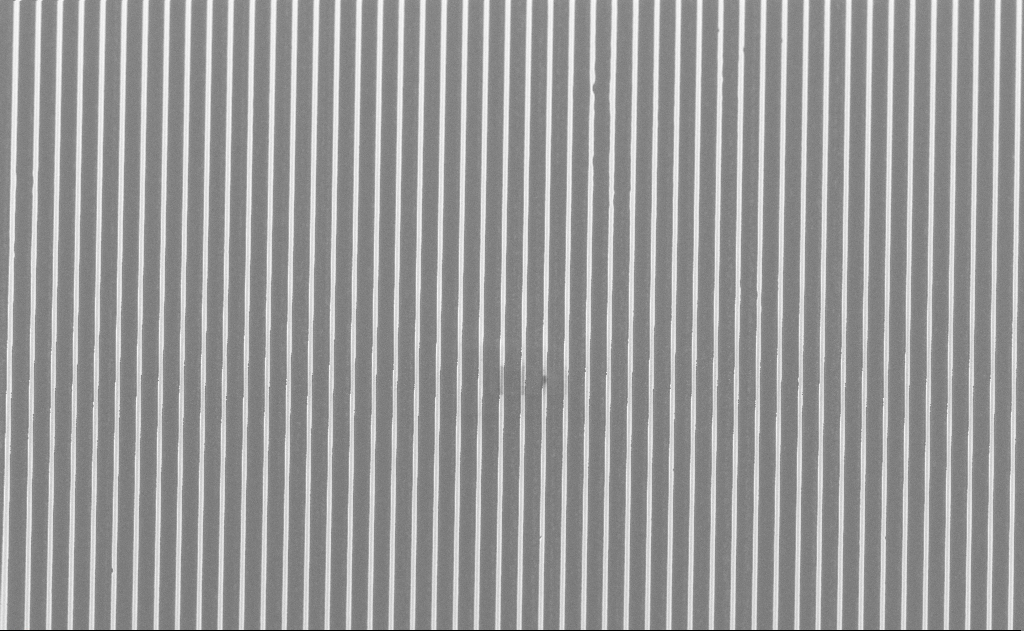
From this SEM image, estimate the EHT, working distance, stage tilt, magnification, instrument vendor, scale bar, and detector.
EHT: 5 kV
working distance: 13 mm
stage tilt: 55°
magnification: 1.96 K X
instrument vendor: Zeiss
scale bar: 10000 nm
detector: SE2